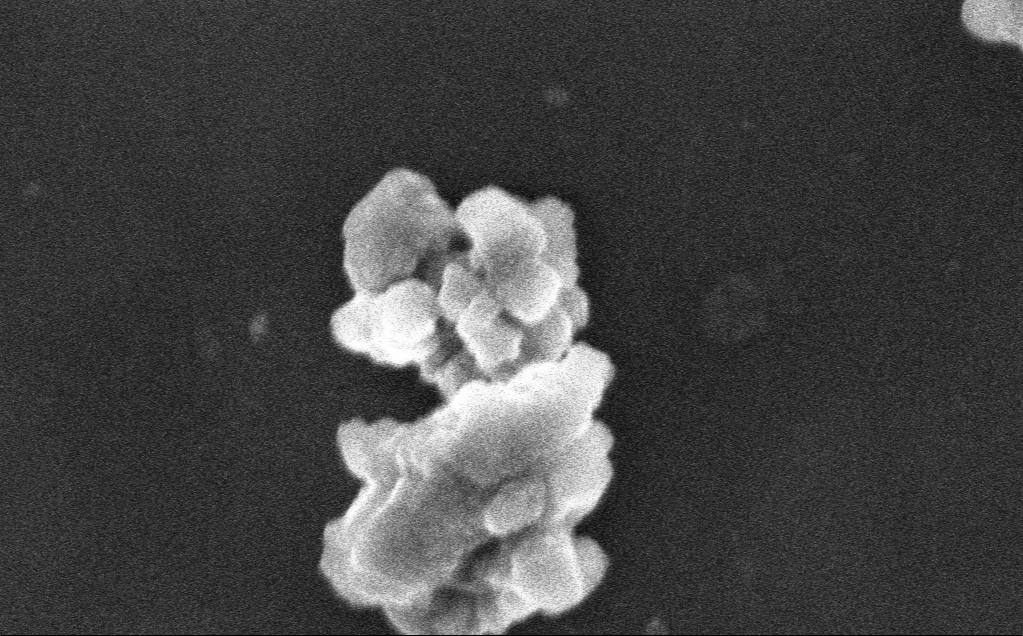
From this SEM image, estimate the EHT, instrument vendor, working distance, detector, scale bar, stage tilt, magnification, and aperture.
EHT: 3 kV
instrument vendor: Zeiss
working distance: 3 mm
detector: InLens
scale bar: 200 nm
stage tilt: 0°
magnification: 215.21 K X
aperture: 30 µm